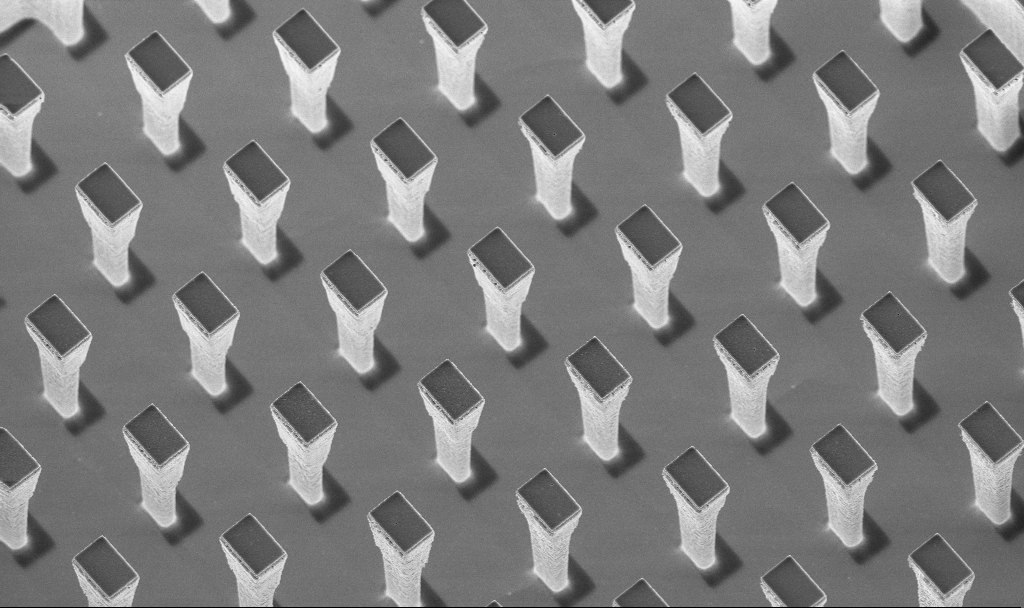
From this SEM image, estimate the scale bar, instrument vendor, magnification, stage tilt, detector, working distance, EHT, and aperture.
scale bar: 10000 nm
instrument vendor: Zeiss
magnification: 4.68 K X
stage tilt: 20°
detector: InLens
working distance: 4.3 mm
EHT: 5 kV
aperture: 30 µm